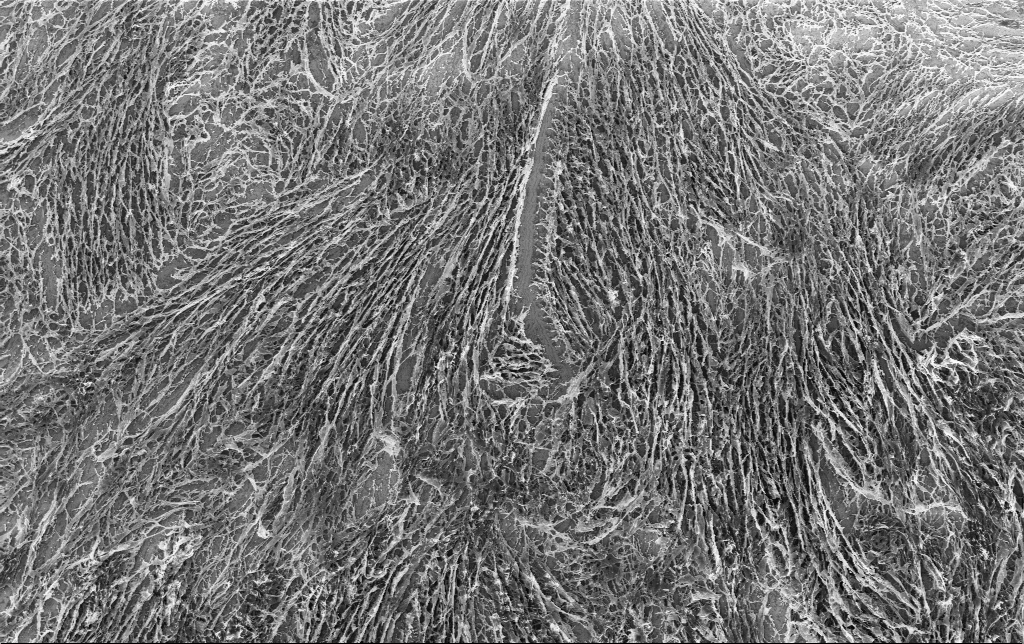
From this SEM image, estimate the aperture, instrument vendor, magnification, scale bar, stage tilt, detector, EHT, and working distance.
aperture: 30 µm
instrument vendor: Zeiss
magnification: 1.53 K X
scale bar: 20000 nm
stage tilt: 0°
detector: InLens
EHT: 3 kV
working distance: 3.4 mm